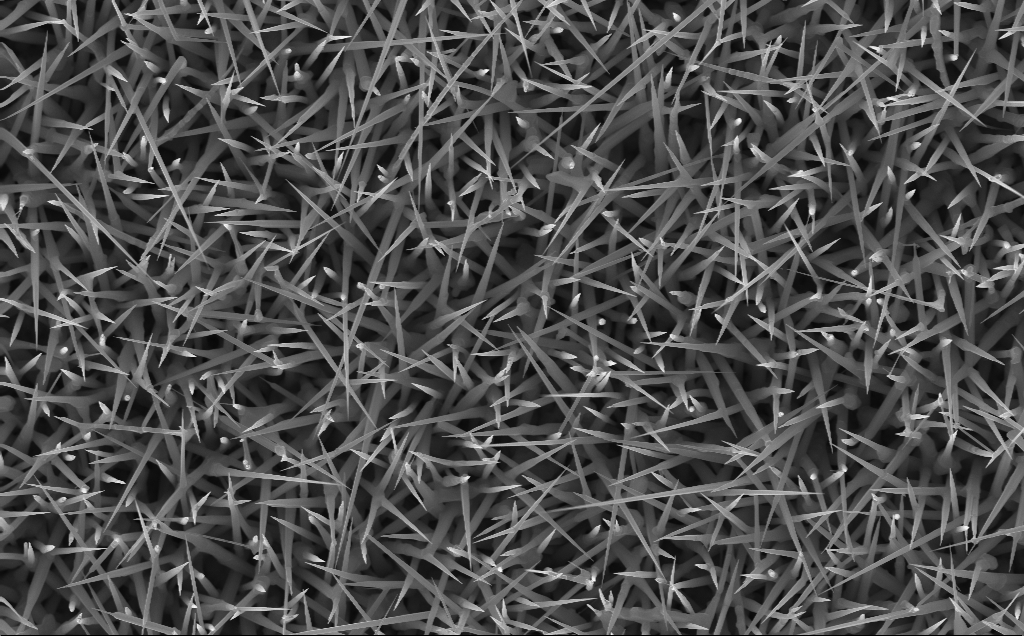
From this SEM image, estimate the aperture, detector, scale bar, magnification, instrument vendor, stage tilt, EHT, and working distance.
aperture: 30 µm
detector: InLens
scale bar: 2000 nm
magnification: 20 K X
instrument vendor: Zeiss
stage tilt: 0°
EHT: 10 kV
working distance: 5 mm